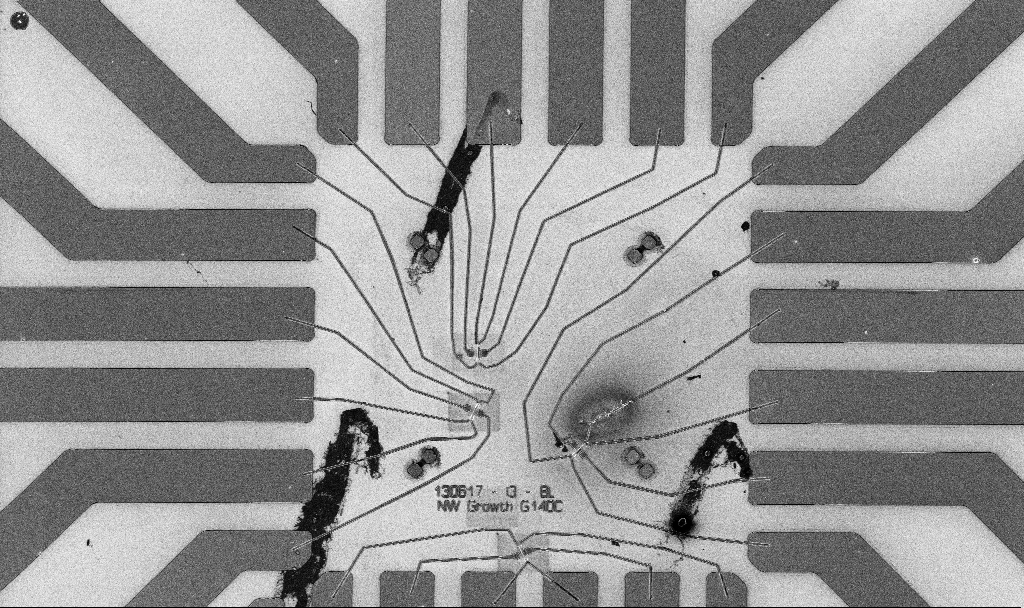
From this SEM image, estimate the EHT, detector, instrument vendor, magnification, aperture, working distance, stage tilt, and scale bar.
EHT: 5 kV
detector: InLens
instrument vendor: Zeiss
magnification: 1 K X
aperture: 30 µm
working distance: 10.7 mm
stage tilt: -0°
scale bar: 20000 nm